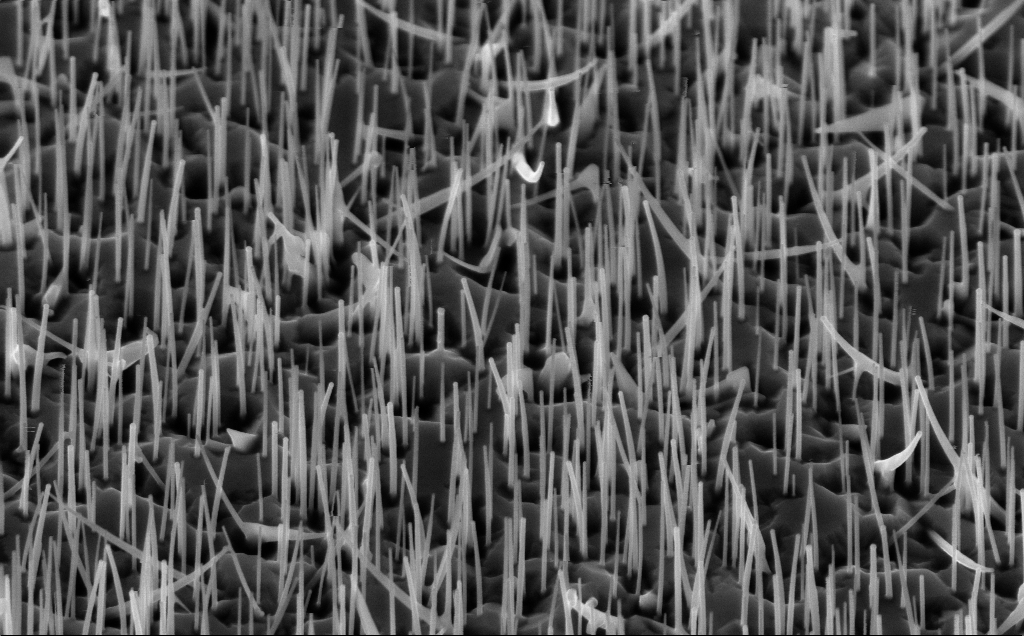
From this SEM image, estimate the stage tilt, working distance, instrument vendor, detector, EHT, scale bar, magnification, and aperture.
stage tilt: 45°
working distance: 5 mm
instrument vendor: Zeiss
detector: InLens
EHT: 10 kV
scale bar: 1000 nm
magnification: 40 K X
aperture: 30 µm